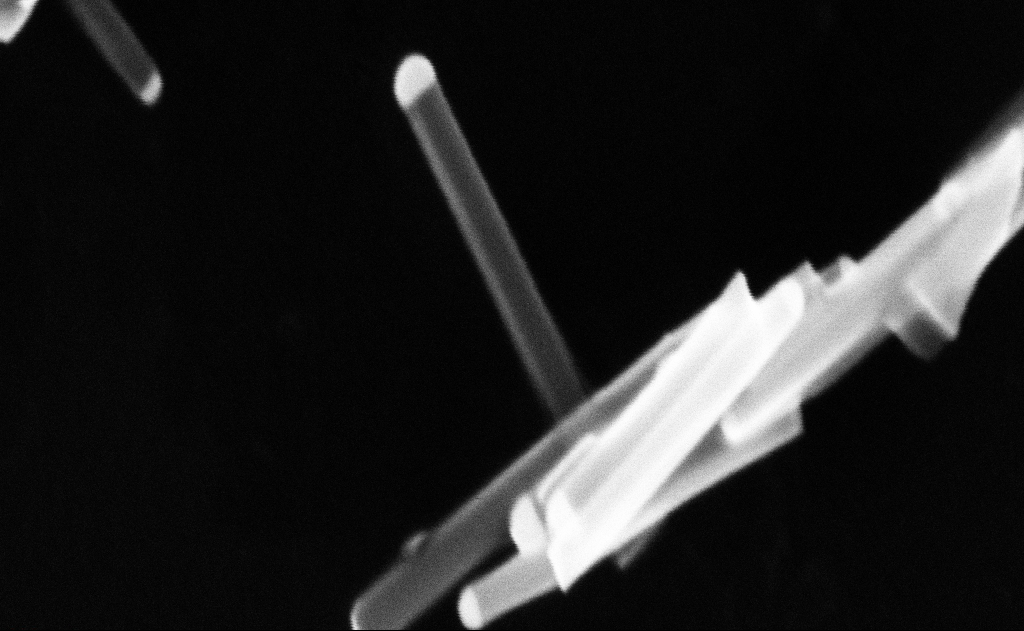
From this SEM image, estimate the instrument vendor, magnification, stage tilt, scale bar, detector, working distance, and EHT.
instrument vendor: Zeiss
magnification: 249.1 K X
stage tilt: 0°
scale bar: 200 nm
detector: InLens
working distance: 11 mm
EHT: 20 kV